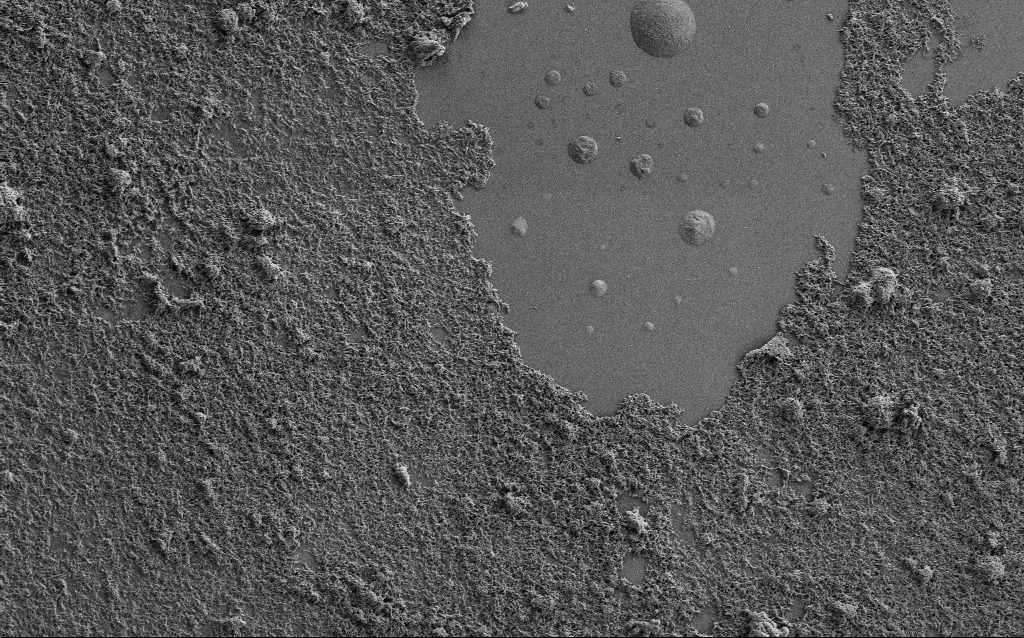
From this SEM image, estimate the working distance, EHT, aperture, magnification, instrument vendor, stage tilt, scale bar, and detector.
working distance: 6 mm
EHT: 1 kV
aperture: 30 µm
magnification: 5 K X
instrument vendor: Zeiss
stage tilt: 0°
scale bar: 10000 nm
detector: SE2